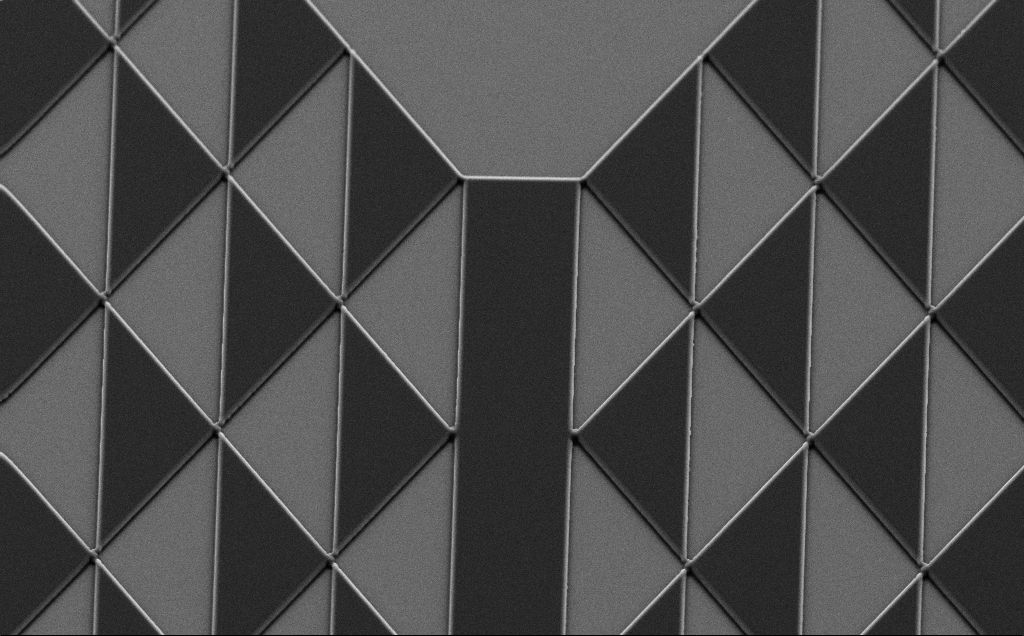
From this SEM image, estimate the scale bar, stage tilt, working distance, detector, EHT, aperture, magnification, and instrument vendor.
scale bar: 10000 nm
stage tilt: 35°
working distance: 8 mm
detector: SE2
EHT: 10 kV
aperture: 30 µm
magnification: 1.23 K X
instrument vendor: Zeiss